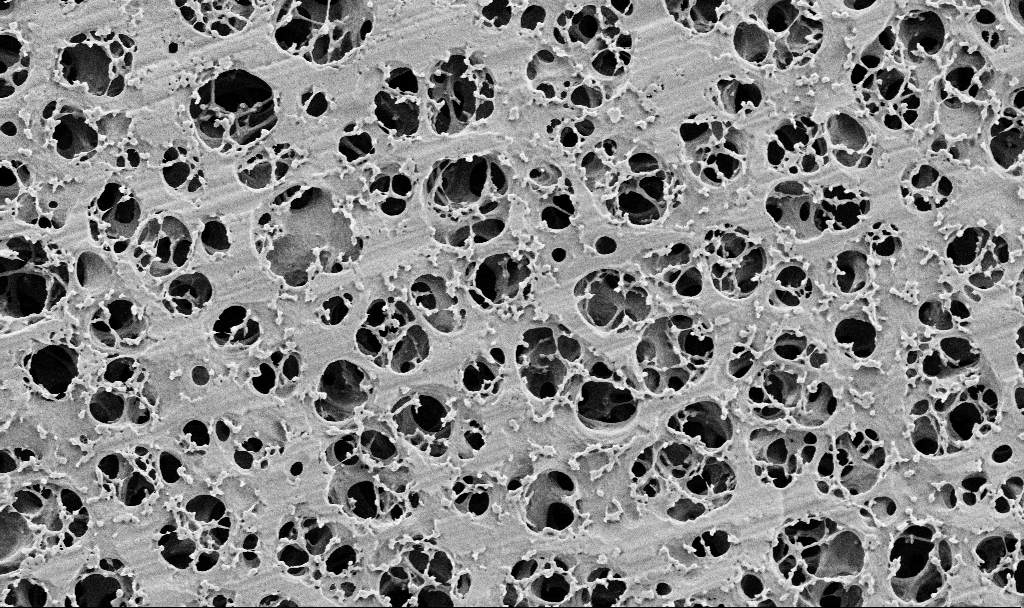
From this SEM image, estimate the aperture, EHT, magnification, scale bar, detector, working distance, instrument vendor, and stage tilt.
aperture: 30 µm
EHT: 2 kV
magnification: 10 K X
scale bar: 2000 nm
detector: SE2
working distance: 3.5 mm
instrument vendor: Zeiss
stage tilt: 0°